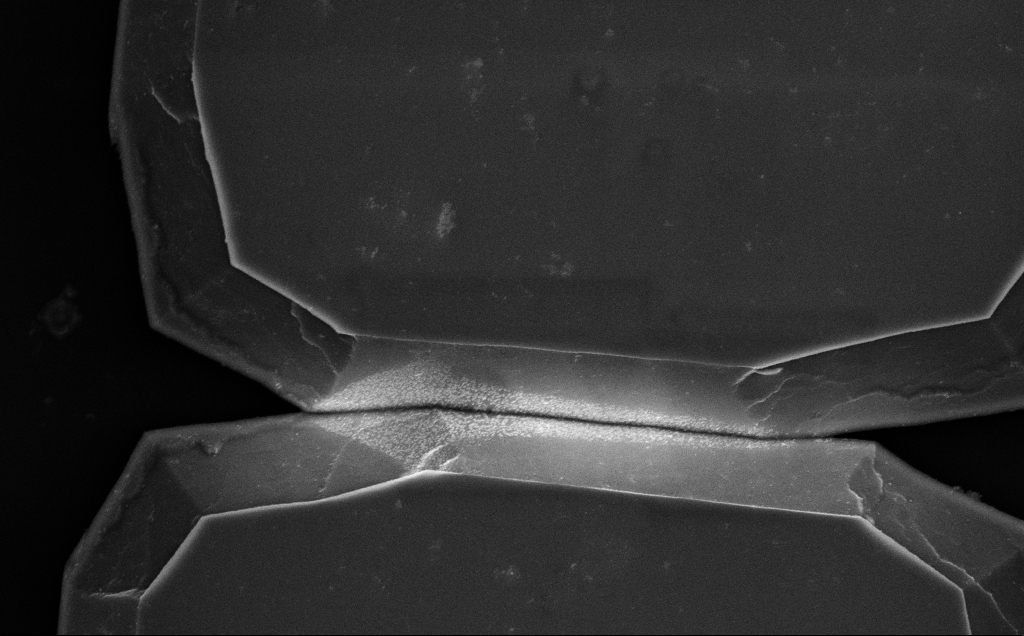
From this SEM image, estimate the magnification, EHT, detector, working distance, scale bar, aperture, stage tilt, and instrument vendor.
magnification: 16.76 K X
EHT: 5 kV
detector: InLens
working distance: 14 mm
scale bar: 2000 nm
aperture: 30 µm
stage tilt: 0°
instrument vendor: Zeiss